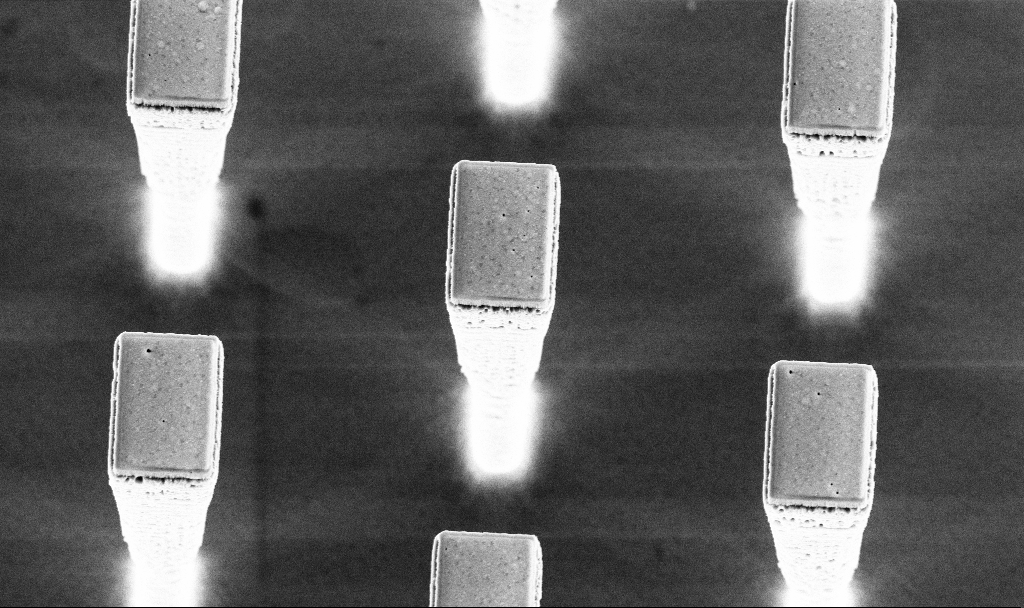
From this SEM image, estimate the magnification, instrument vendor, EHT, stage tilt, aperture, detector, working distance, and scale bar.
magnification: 12.01 K X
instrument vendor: Zeiss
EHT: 5 kV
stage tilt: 20.2°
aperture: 30 µm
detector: InLens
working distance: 4.1 mm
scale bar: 2000 nm